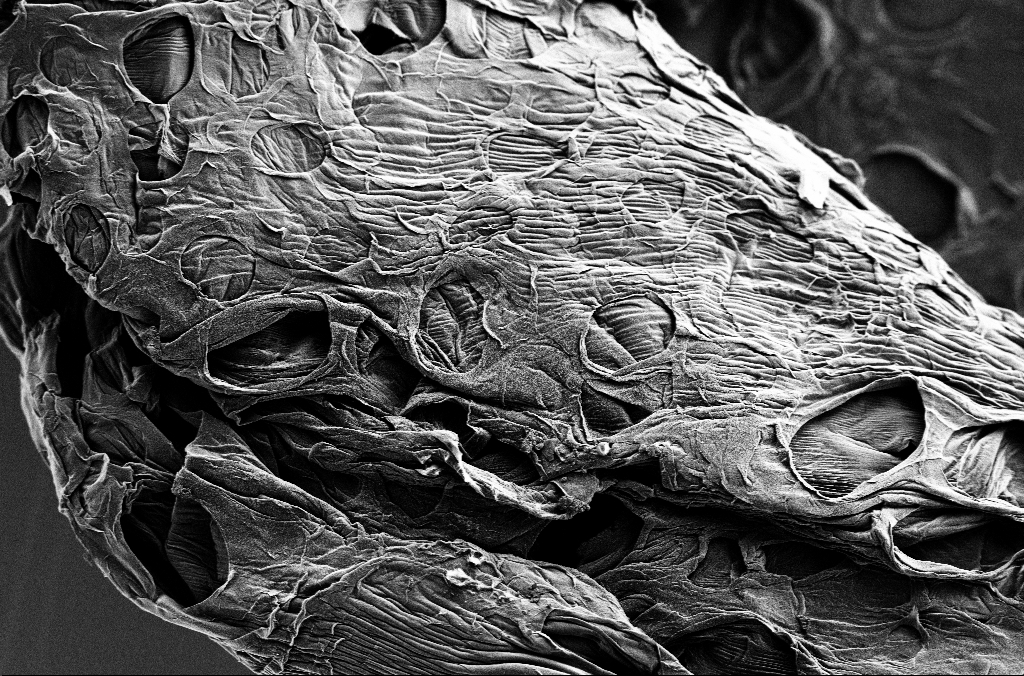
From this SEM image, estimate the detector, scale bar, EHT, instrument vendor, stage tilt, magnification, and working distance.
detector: SE2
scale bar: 20000 nm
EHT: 1.8 kV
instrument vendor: Zeiss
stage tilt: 0°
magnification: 1 K X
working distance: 5.4 mm